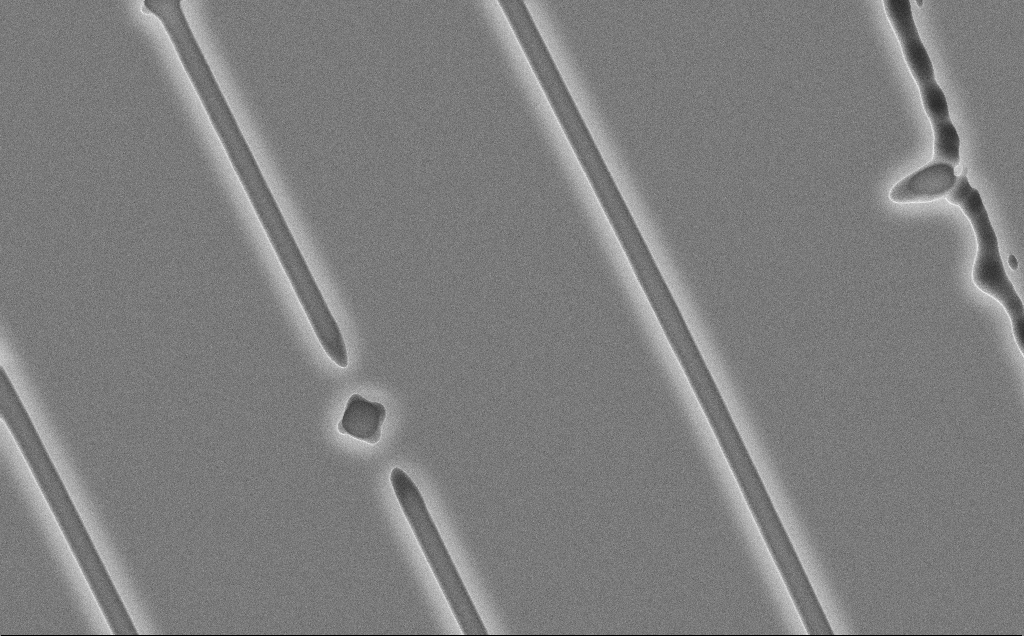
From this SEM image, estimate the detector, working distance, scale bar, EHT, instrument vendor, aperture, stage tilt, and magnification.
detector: SE2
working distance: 12 mm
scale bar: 10000 nm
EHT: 10 kV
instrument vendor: Zeiss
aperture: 30 µm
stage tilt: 0°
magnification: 5.69 K X